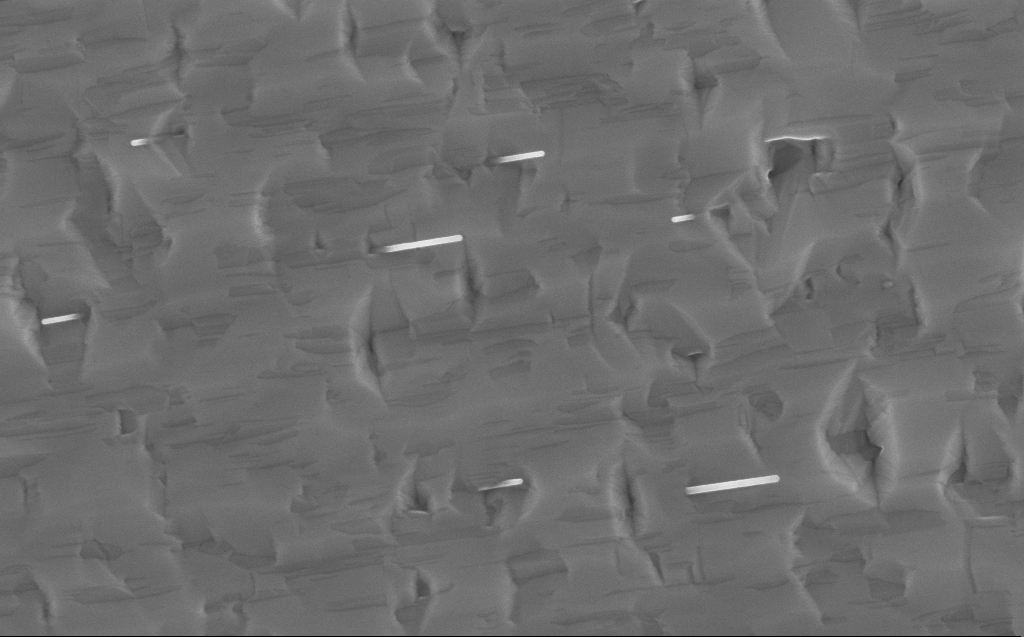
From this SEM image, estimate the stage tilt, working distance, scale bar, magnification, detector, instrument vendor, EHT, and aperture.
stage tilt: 0°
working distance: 3 mm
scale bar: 200 nm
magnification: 80 K X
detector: InLens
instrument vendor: Zeiss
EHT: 10 kV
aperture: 30 µm